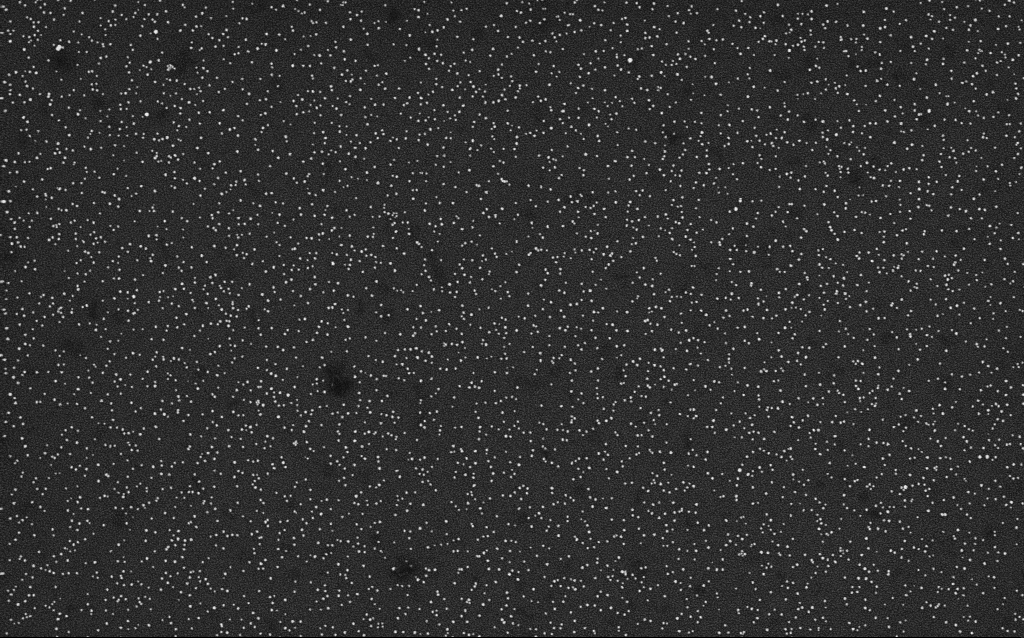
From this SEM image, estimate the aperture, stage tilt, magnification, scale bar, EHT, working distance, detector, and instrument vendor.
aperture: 30 µm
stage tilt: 0°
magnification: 50 K X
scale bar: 1000 nm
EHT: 4 kV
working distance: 2.1 mm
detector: InLens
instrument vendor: Zeiss